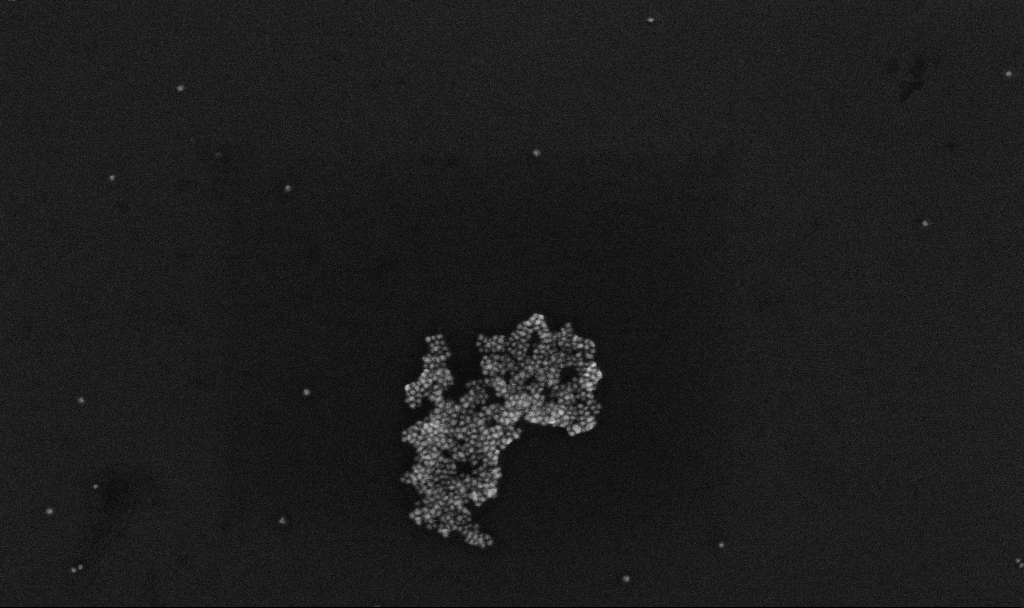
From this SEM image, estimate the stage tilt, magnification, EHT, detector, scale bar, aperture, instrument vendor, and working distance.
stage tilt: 0°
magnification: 100 K X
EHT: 10 kV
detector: InLens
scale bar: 200 nm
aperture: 30 µm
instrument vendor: Zeiss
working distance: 3.3 mm